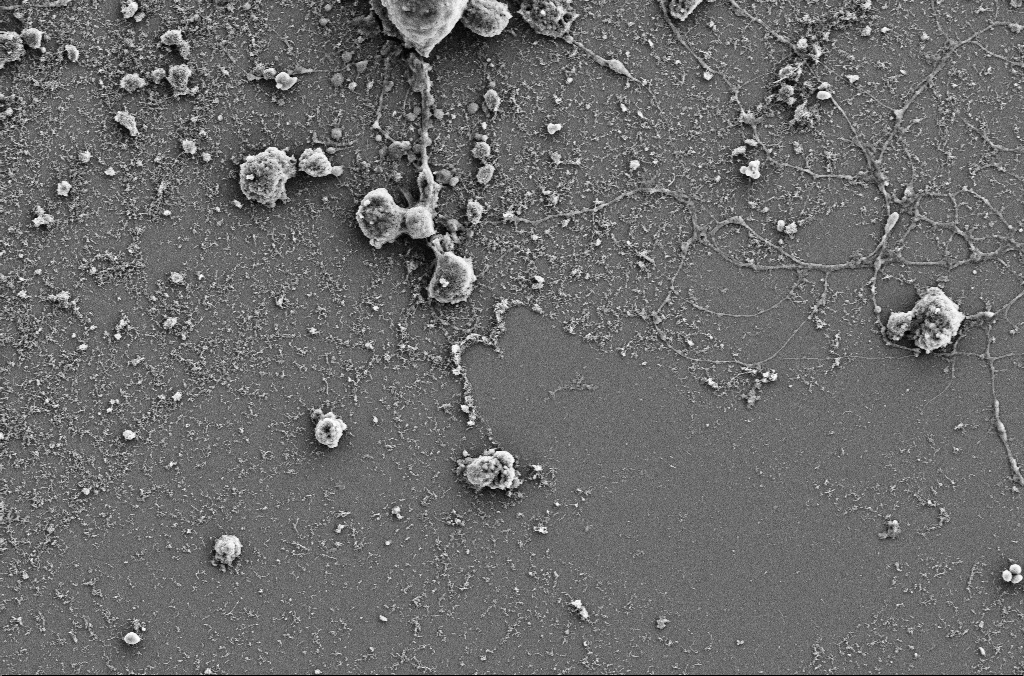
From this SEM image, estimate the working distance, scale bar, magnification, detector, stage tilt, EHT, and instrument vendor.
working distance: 4 mm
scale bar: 10000 nm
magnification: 4 K X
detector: SE2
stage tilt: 0°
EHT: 5 kV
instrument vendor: Zeiss